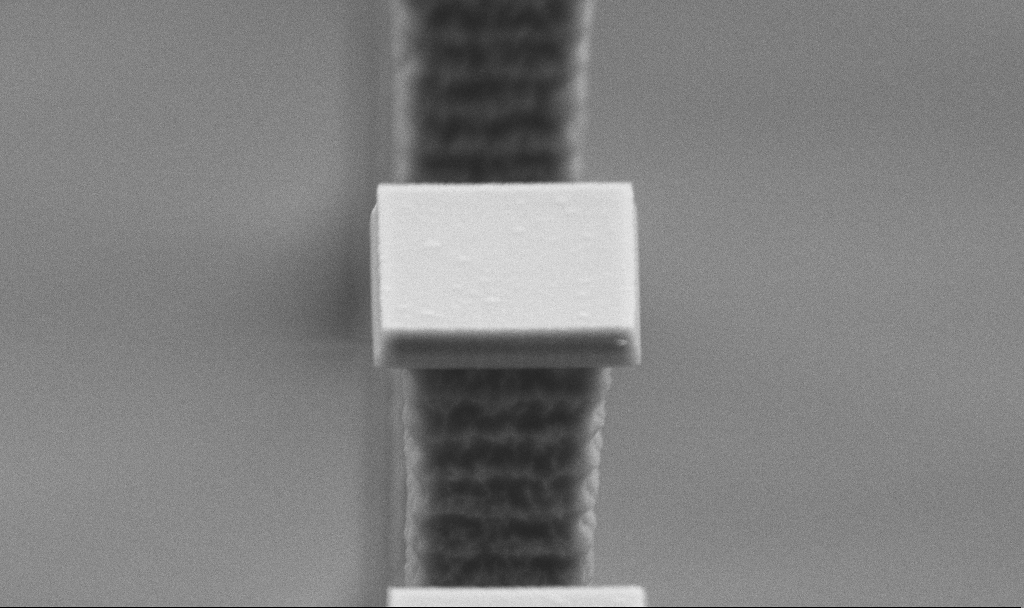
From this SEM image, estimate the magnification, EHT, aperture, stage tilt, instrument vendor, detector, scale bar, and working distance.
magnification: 32.42 K X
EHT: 5 kV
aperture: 30 µm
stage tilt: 70°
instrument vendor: Zeiss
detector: SE2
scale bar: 2000 nm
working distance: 5.6 mm